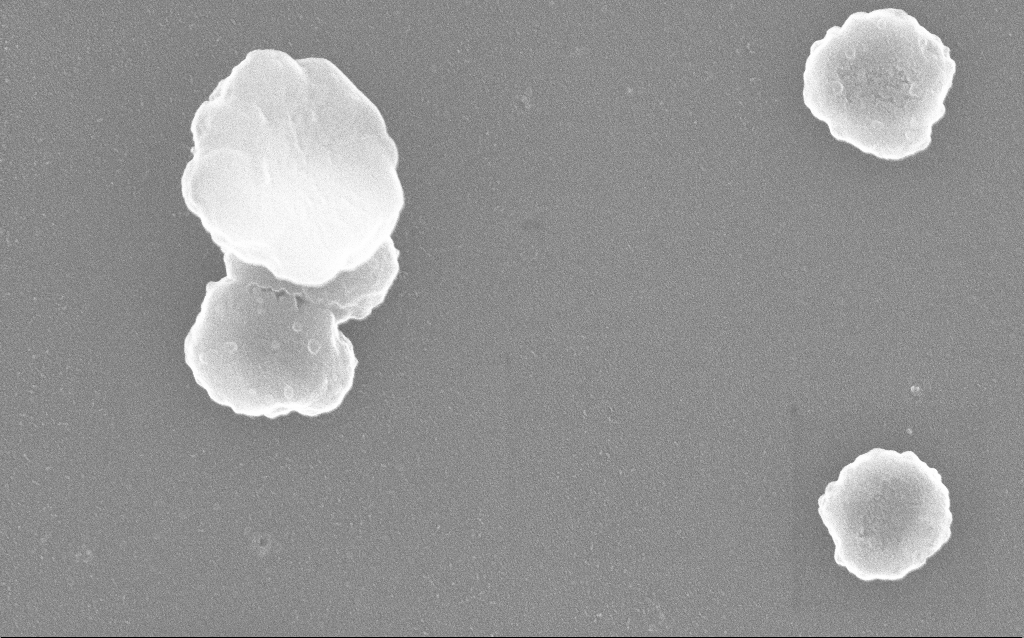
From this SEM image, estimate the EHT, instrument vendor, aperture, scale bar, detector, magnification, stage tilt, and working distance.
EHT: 20 kV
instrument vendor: Zeiss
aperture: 30 µm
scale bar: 200 nm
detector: InLens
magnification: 100 K X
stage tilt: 0°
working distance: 1.4 mm